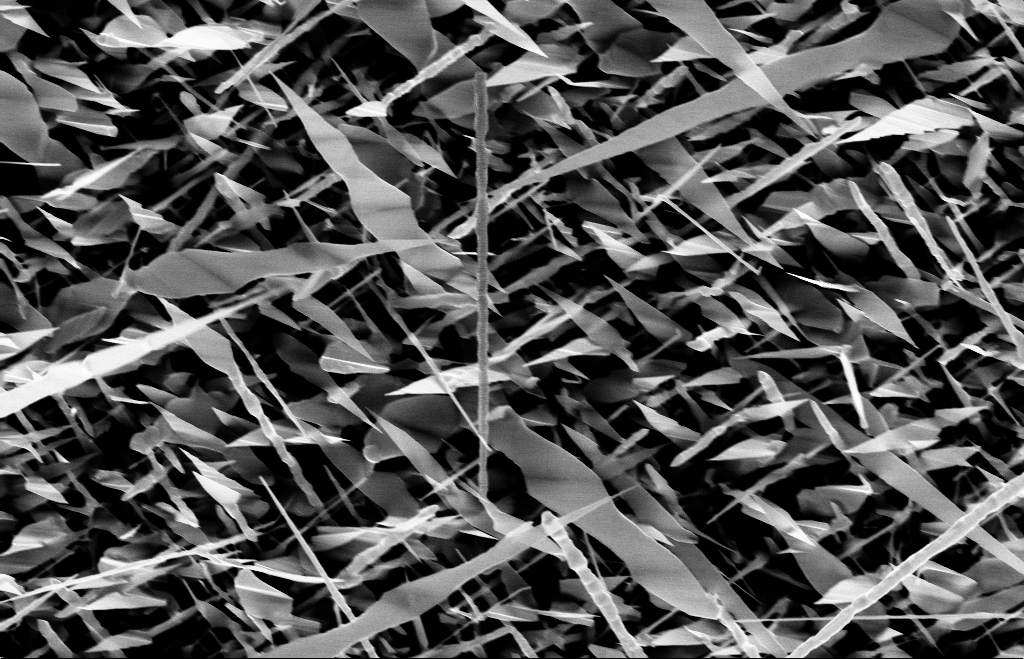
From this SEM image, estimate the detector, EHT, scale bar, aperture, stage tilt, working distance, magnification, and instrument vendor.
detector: InLens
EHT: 10 kV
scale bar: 1000 nm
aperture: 30 µm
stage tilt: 0°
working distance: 8 mm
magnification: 20 K X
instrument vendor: Zeiss